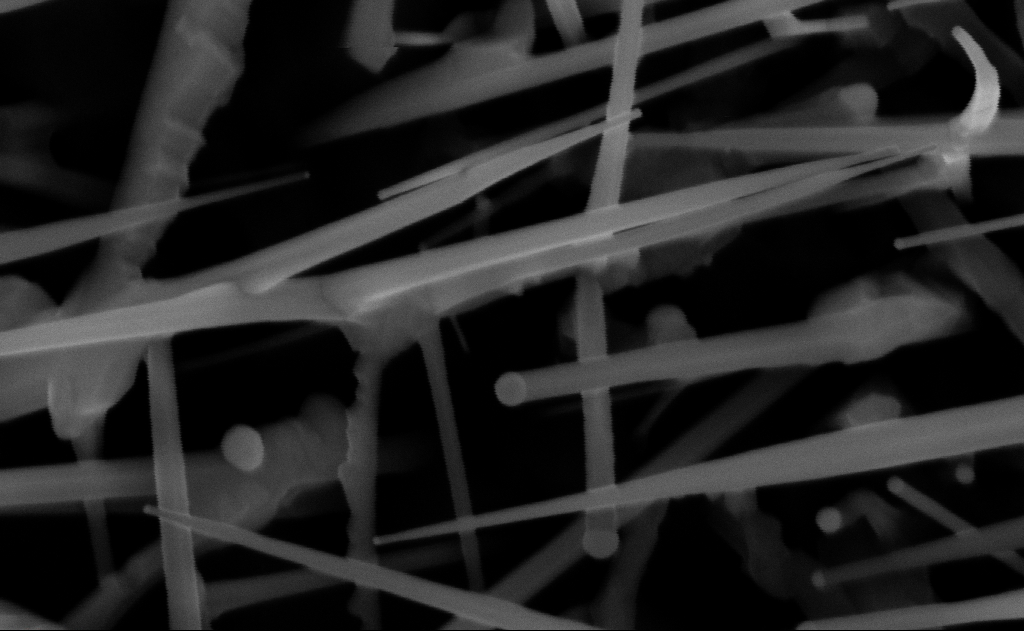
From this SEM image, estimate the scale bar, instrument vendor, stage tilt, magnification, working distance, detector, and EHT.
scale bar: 100 nm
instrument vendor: Zeiss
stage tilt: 0°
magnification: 150 K X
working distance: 15 mm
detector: InLens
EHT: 10 kV